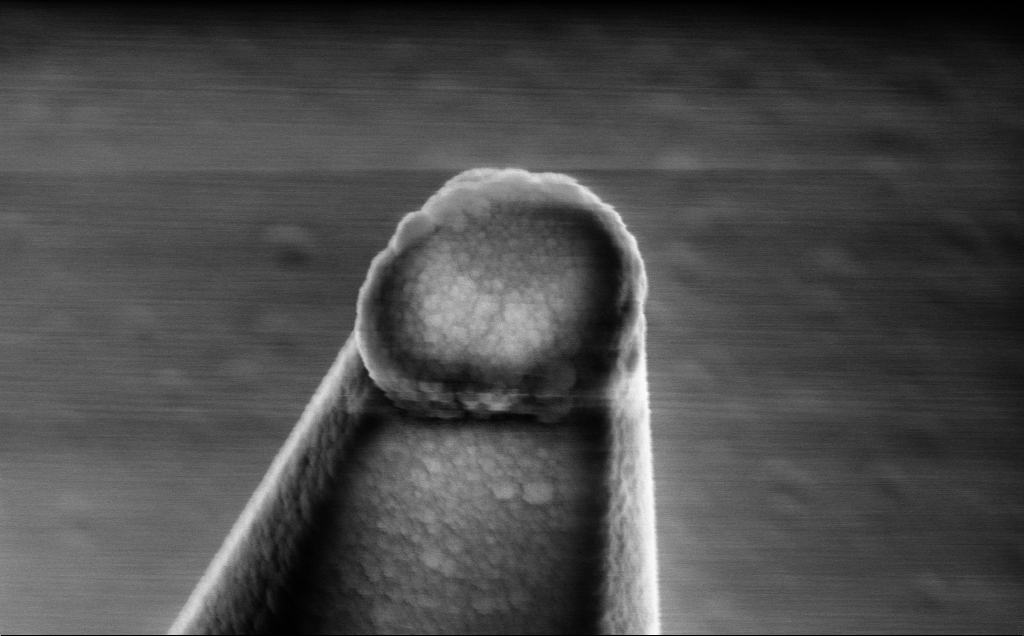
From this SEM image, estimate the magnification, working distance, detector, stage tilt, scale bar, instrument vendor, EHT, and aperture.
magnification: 58.29 K X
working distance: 7 mm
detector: InLens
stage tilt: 0°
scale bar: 1000 nm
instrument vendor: Zeiss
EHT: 5 kV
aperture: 30 µm